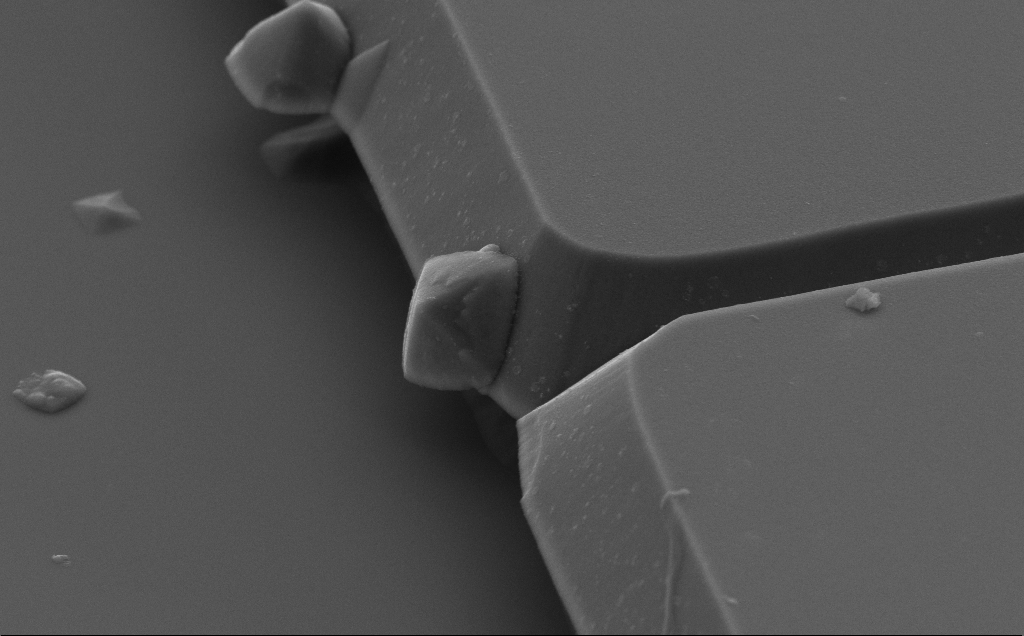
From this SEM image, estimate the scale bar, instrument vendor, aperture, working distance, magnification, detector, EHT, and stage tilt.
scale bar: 2000 nm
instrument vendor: Zeiss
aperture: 30 µm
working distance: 10 mm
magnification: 20.17 K X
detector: SE2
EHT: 5 kV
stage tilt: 50°